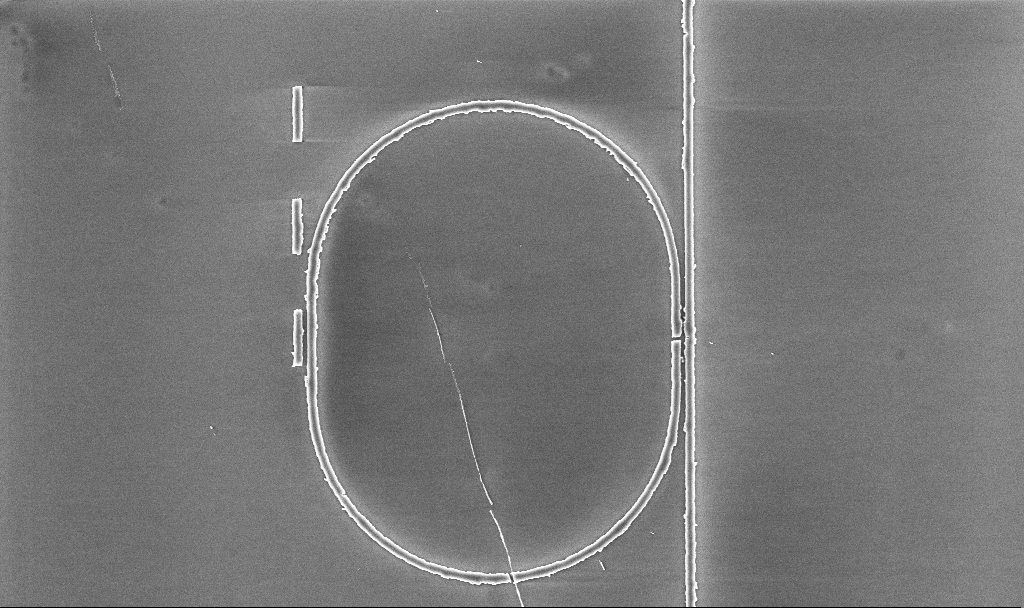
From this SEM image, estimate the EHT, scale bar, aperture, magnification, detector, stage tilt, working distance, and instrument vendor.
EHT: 5 kV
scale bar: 10000 nm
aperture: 30 µm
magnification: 6.94 K X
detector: InLens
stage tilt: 0°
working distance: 5.2 mm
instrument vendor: Zeiss